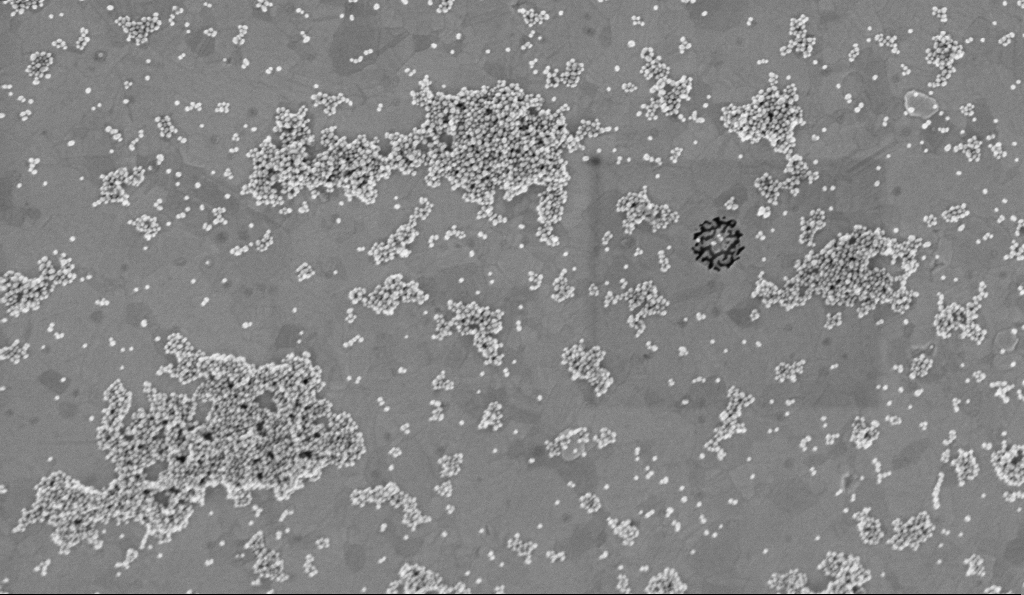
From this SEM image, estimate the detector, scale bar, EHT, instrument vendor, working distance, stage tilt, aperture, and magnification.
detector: InLens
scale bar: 200 nm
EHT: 2 kV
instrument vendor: Zeiss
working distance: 3.2 mm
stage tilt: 0°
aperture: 30 µm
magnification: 75 K X